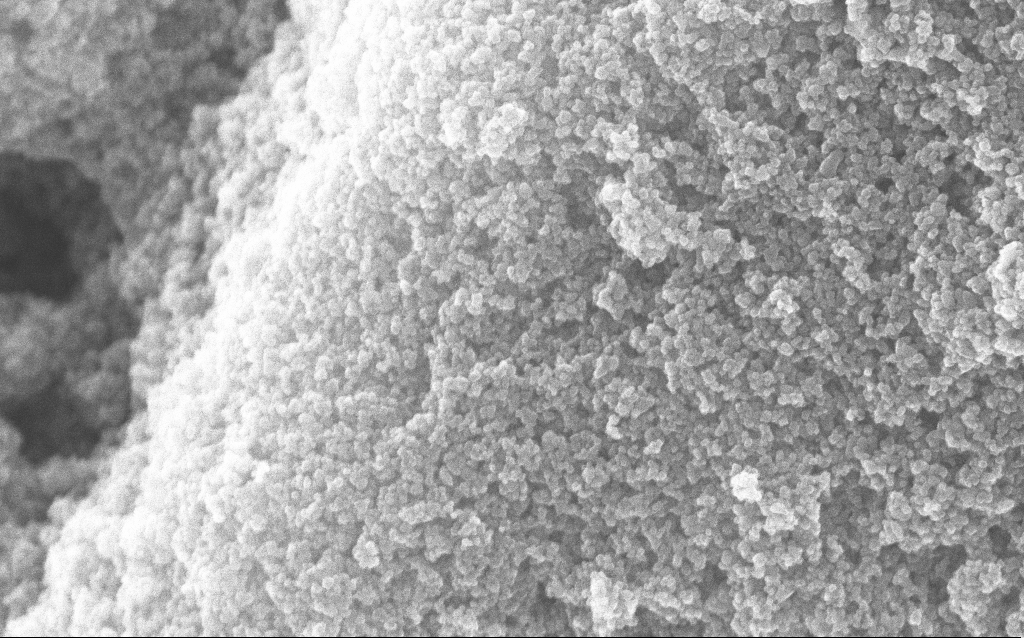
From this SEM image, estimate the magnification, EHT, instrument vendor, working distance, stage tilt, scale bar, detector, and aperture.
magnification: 121.23 K X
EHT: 10 kV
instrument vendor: Zeiss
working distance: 3.6 mm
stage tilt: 0°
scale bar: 200 nm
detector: InLens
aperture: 30 µm